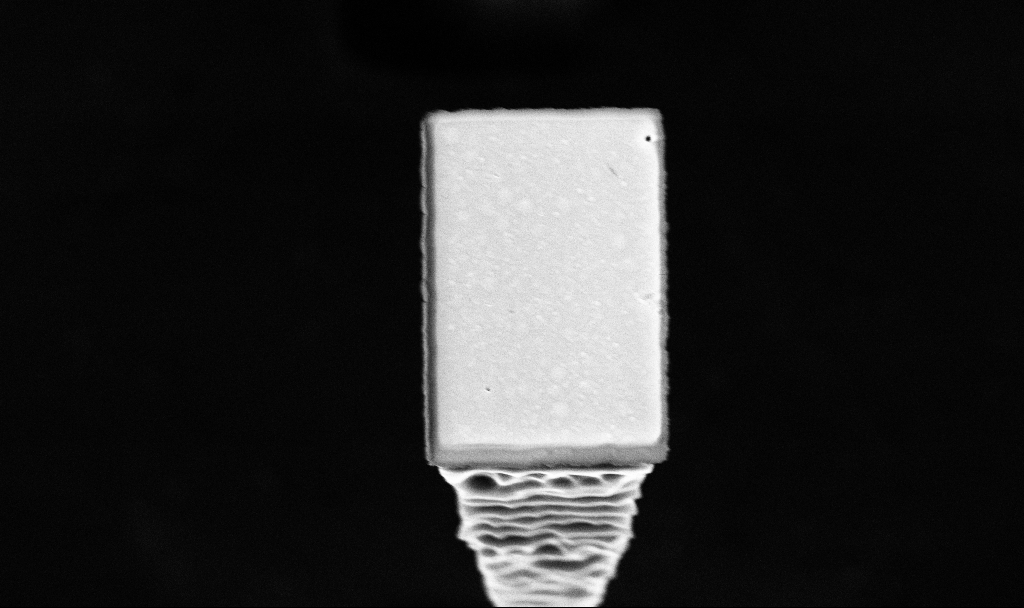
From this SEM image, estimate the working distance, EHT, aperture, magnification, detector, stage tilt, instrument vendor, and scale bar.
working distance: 4.3 mm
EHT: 5 kV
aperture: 30 µm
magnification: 29.51 K X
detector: InLens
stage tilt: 20°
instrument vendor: Zeiss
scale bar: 1000 nm